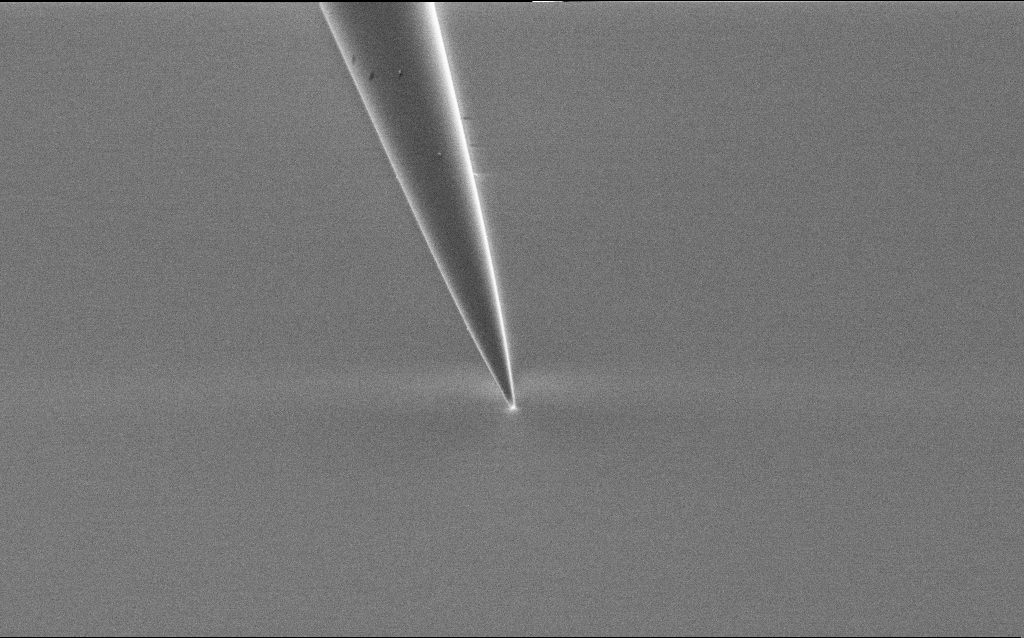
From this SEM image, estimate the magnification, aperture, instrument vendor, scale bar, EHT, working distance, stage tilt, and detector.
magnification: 10 K X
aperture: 30 µm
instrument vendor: Zeiss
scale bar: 2000 nm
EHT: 1 kV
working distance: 7 mm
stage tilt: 45°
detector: SE2